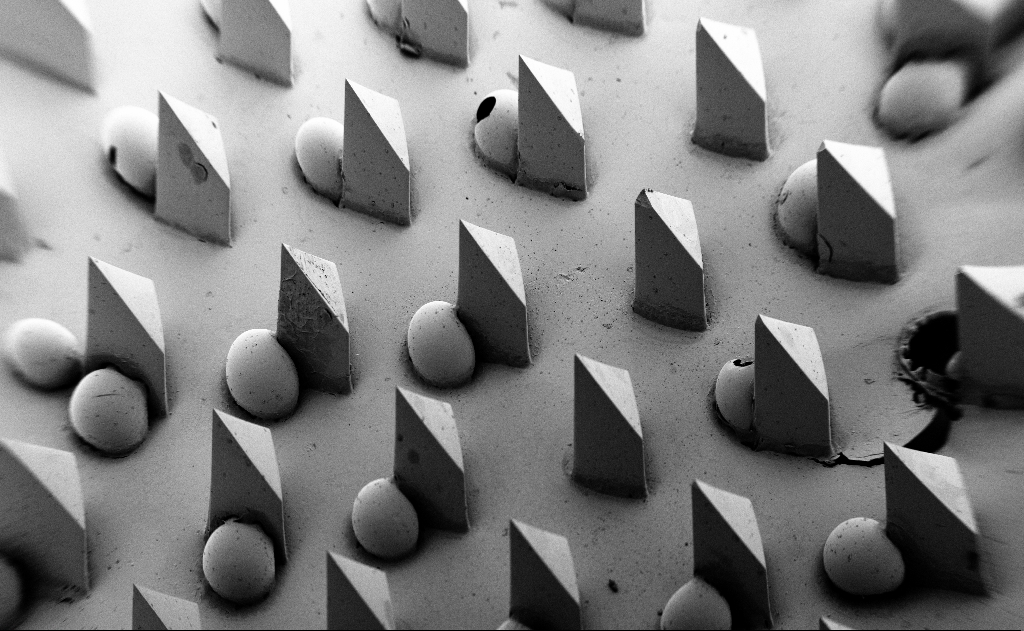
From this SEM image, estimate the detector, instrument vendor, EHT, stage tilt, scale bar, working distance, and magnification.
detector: SE2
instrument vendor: Zeiss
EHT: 5 kV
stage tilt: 45°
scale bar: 1e+06 nm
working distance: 9 mm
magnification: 0.067 K X